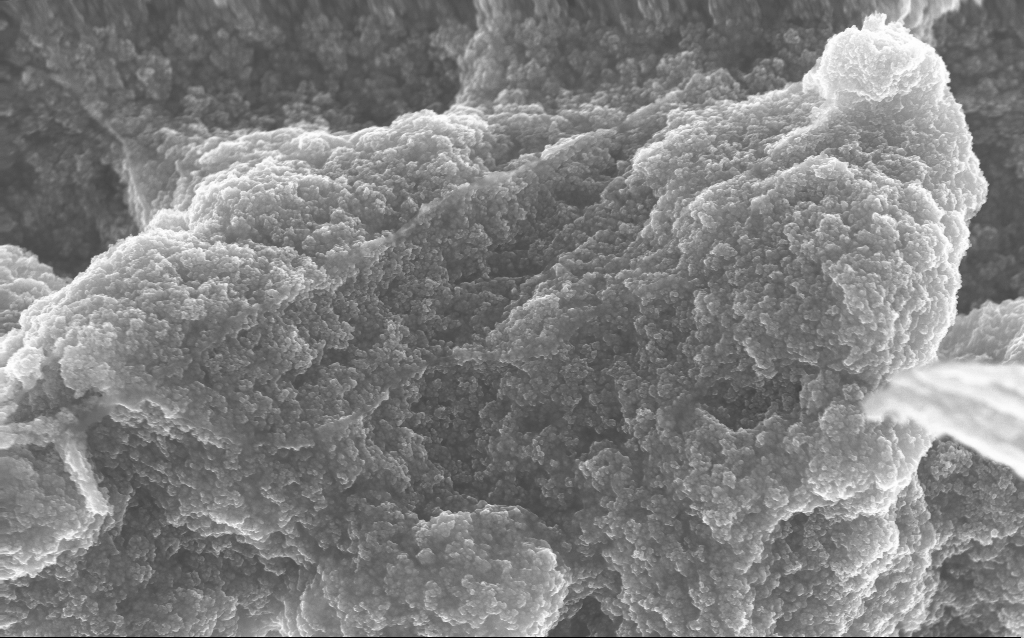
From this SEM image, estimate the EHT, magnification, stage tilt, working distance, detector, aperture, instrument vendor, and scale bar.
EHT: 10 kV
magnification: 37.88 K X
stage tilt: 0°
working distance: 2.8 mm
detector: InLens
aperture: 30 µm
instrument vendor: Zeiss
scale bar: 1000 nm